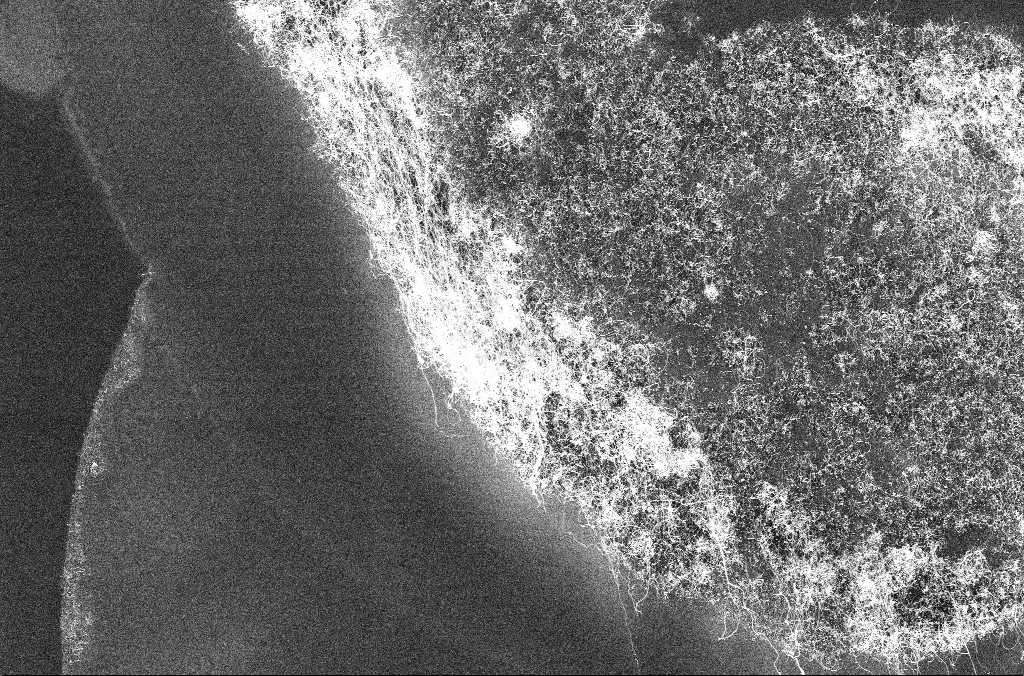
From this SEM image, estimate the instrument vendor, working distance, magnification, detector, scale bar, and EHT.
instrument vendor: Zeiss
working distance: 3.3 mm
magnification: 1.97 K X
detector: InLens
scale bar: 10000 nm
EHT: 10 kV